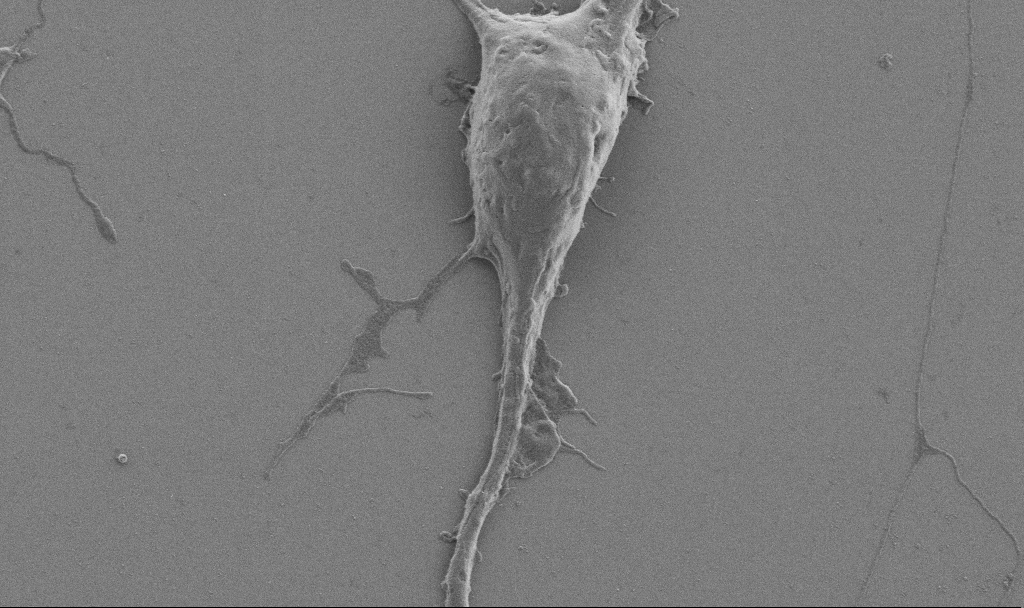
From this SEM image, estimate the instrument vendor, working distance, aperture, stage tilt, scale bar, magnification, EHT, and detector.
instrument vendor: Zeiss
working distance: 6.9 mm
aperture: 30 µm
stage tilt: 0°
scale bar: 2000 nm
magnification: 10 K X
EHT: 0.9 kV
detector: SE2